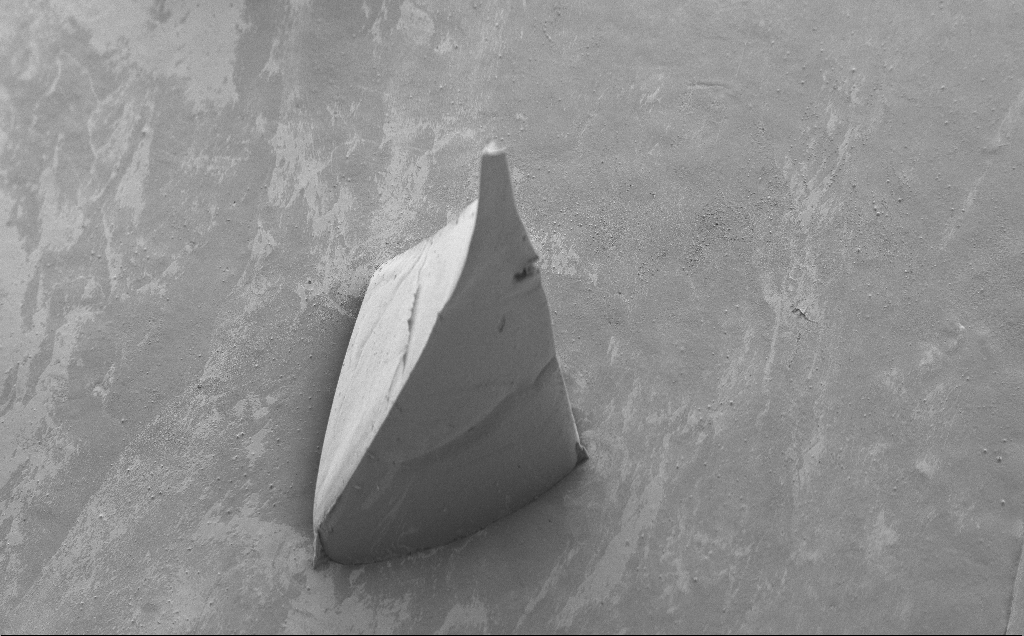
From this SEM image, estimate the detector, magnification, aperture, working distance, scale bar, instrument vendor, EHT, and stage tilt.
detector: SE2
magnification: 0.154 K X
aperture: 30 µm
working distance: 8 mm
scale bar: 200000 nm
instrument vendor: Zeiss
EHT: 5 kV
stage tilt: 40°